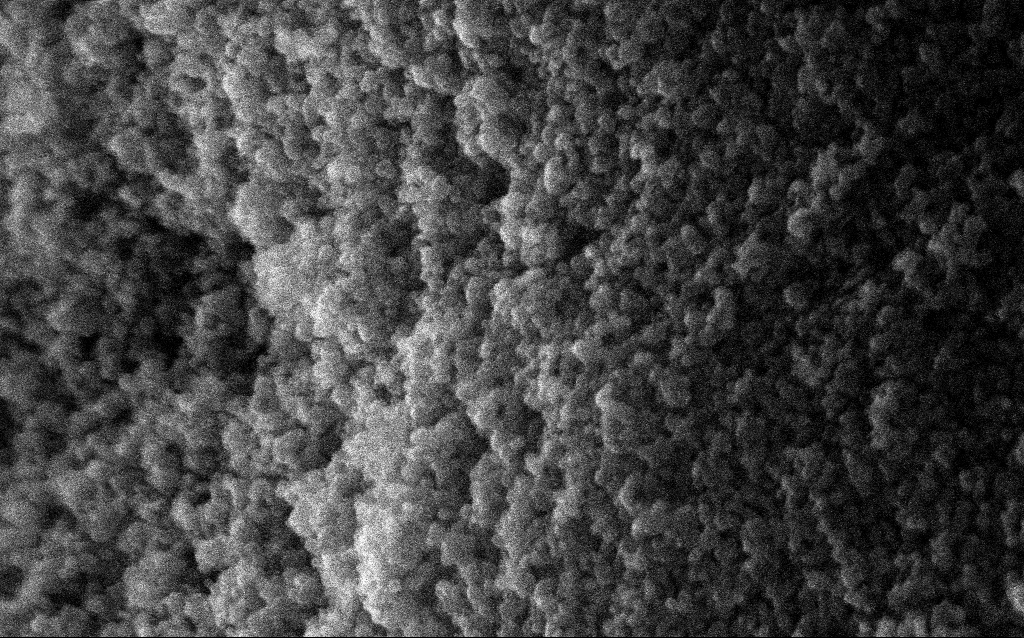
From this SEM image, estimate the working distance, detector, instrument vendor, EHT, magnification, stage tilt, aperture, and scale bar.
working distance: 2.7 mm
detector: InLens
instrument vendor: Zeiss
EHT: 10 kV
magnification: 211.33 K X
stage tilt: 0°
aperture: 30 µm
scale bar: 100 nm